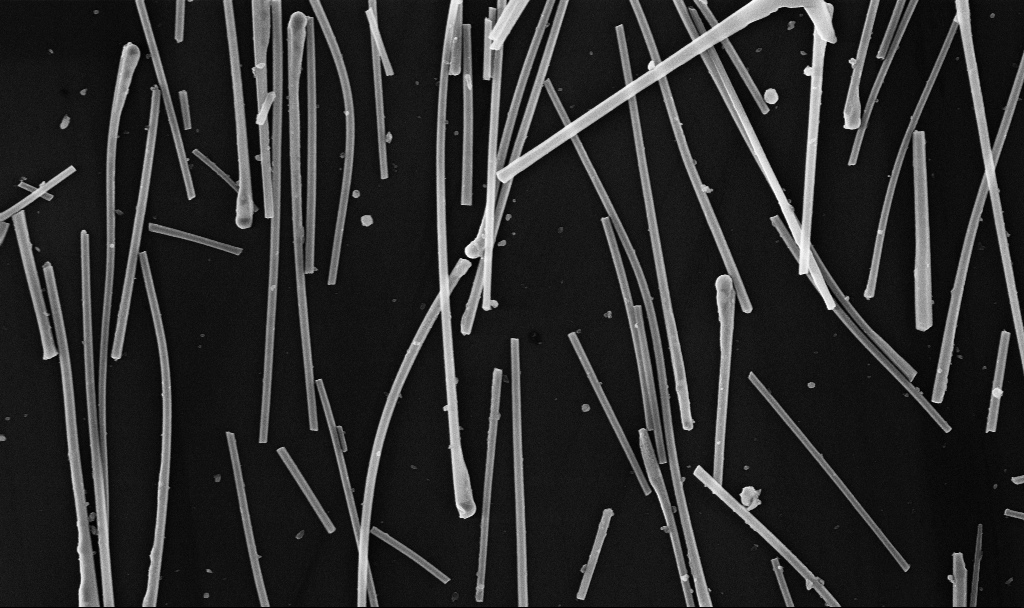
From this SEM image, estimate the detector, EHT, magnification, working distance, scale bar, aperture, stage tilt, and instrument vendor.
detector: InLens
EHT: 10 kV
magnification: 30 K X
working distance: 6.7 mm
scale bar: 1000 nm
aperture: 30 µm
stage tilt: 0°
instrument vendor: Zeiss